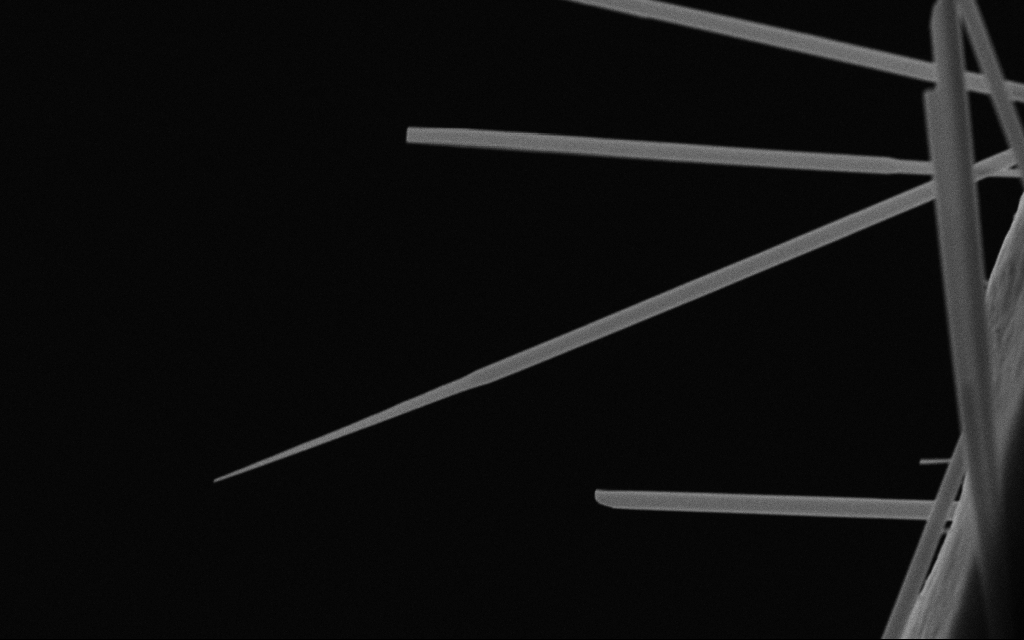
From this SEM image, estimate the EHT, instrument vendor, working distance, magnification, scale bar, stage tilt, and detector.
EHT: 20 kV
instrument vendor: Zeiss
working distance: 7 mm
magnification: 43.99 K X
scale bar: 1000 nm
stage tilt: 0°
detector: SE2